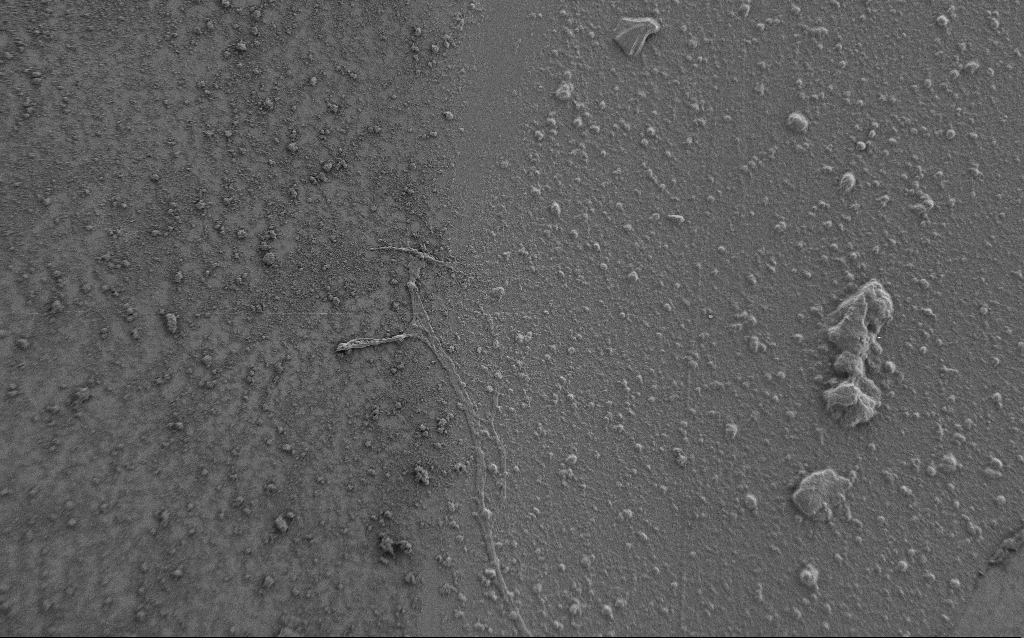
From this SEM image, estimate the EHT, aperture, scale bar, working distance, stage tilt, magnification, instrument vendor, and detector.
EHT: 0.9 kV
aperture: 30 µm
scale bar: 20000 nm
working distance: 7 mm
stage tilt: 0°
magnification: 1 K X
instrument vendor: Zeiss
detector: SE2